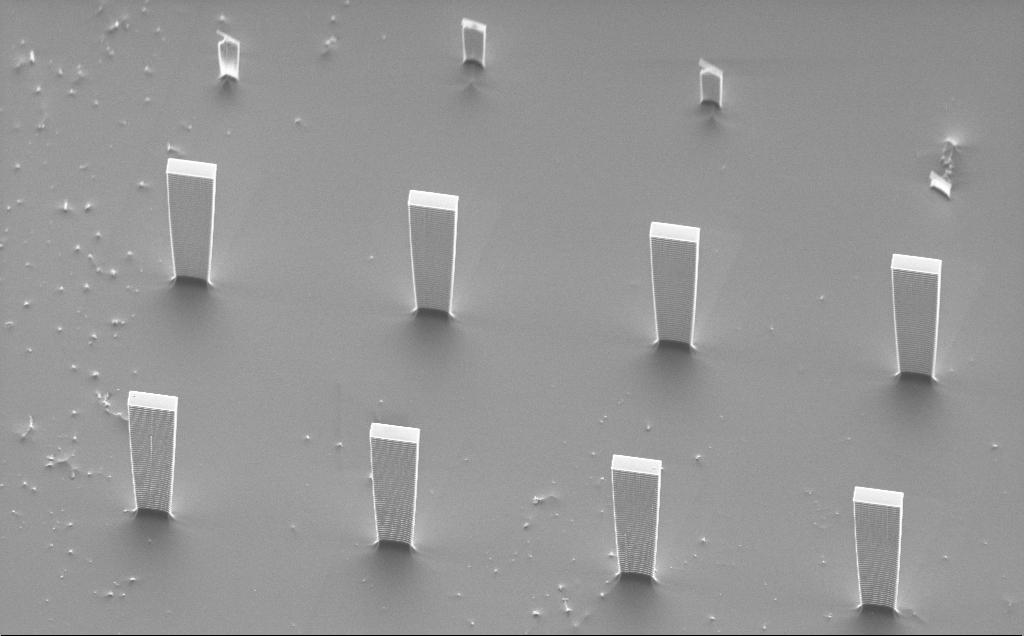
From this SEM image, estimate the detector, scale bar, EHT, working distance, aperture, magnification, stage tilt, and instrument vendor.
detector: SE2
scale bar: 10000 nm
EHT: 5 kV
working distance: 16 mm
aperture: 30 µm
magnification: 1.8 K X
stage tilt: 50°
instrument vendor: Zeiss